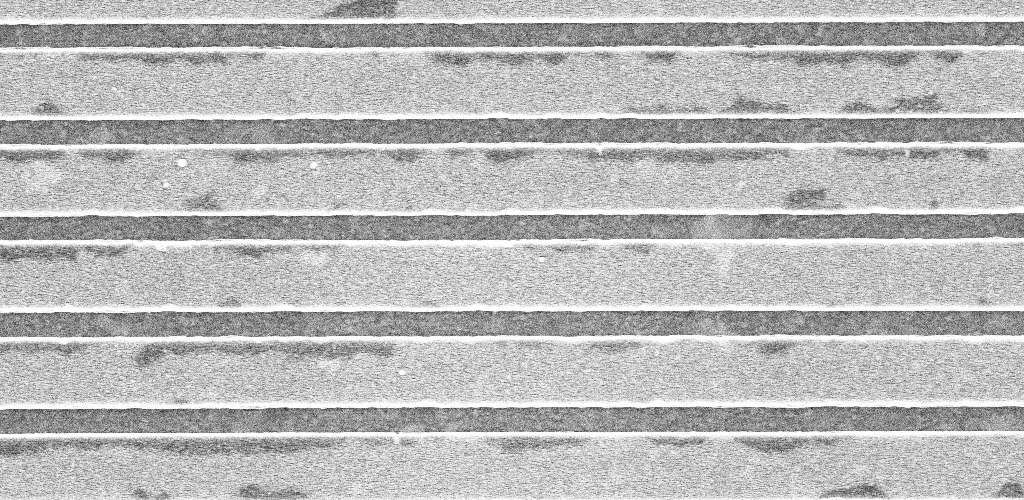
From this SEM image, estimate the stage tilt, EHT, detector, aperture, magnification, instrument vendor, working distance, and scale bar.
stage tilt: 0°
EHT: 5 kV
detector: InLens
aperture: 30 µm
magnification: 44.61 K X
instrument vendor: Zeiss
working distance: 3.1 mm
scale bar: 1000 nm